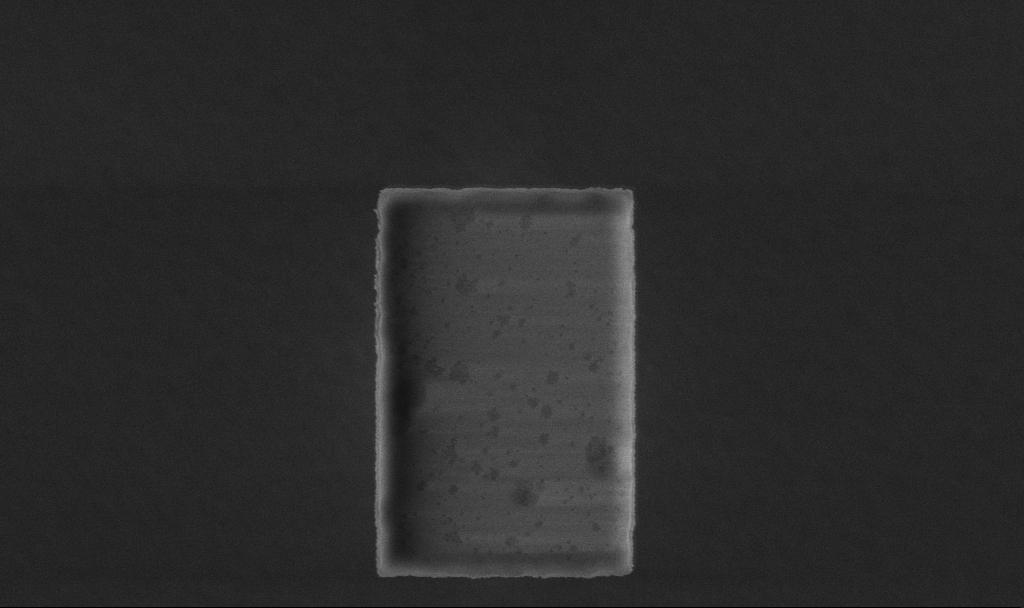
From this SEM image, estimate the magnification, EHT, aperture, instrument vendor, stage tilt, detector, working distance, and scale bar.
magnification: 31.5 K X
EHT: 5 kV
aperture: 30 µm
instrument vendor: Zeiss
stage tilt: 0°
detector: InLens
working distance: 4.7 mm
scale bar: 1000 nm